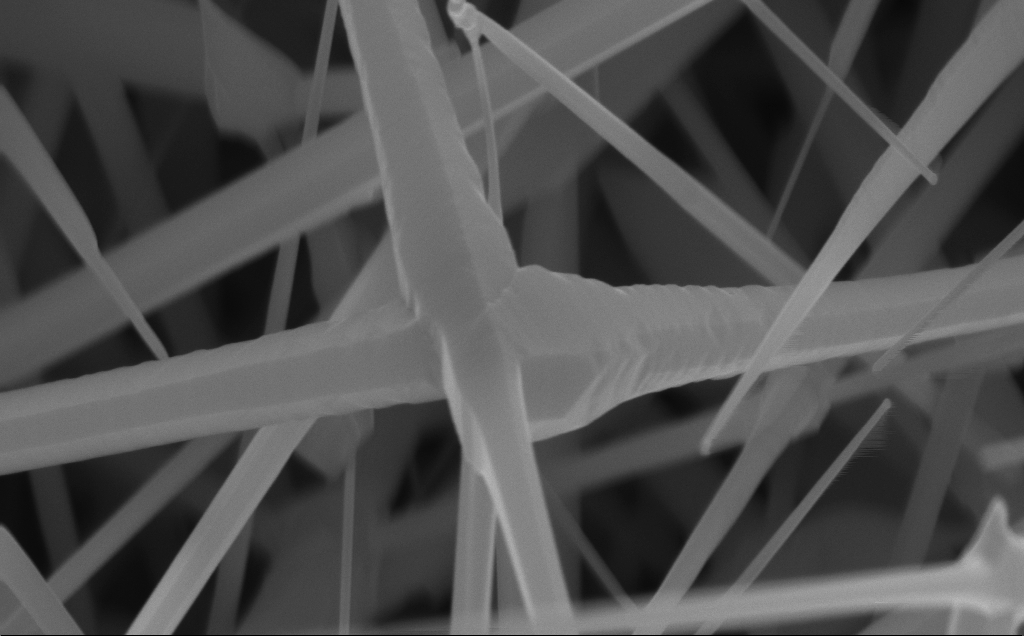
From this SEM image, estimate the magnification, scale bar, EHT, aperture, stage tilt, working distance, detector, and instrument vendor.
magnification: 90 K X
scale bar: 200 nm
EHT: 10 kV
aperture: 30 µm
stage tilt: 0°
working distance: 4 mm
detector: InLens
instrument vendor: Zeiss